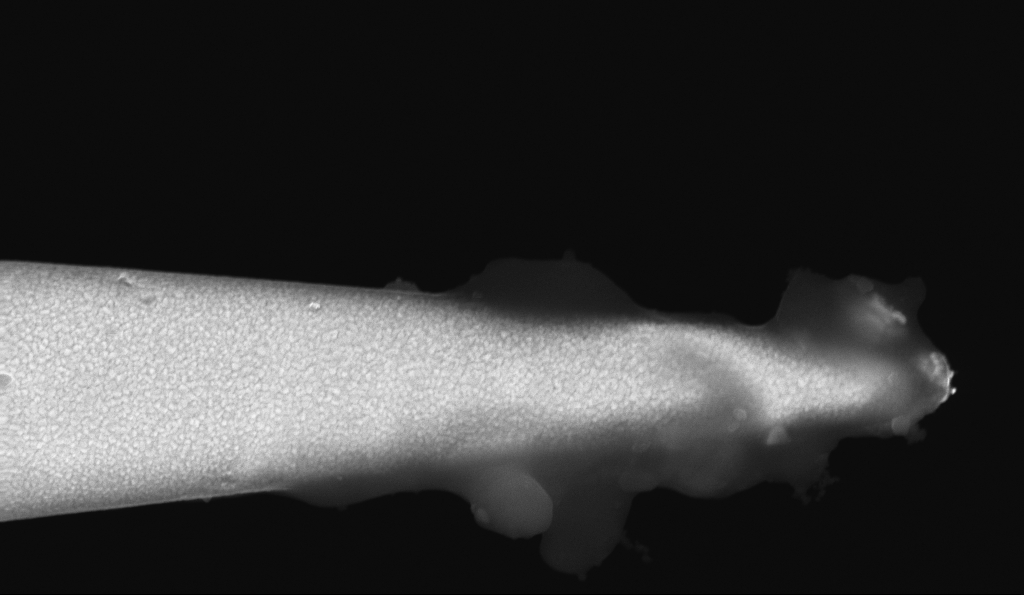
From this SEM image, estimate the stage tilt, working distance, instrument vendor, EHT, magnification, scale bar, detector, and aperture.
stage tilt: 1.3°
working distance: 3.8 mm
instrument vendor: Zeiss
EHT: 10 kV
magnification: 44.6 K X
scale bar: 1000 nm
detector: InLens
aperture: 30 µm